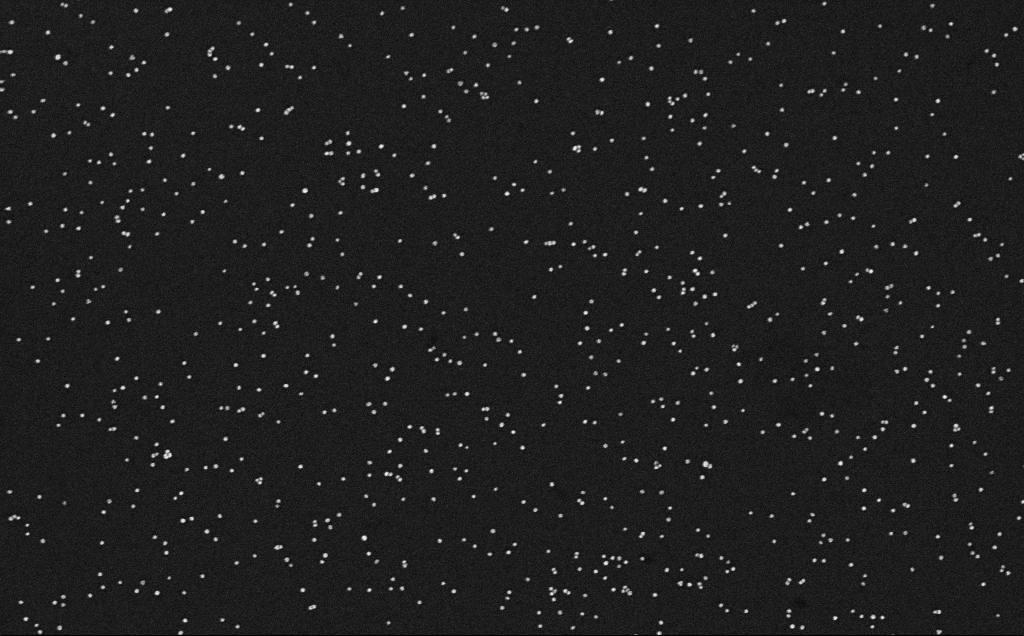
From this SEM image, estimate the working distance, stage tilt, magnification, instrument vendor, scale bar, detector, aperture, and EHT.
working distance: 3.2 mm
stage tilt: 0°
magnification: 100 K X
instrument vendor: Zeiss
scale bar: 200 nm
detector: InLens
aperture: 30 µm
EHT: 10 kV